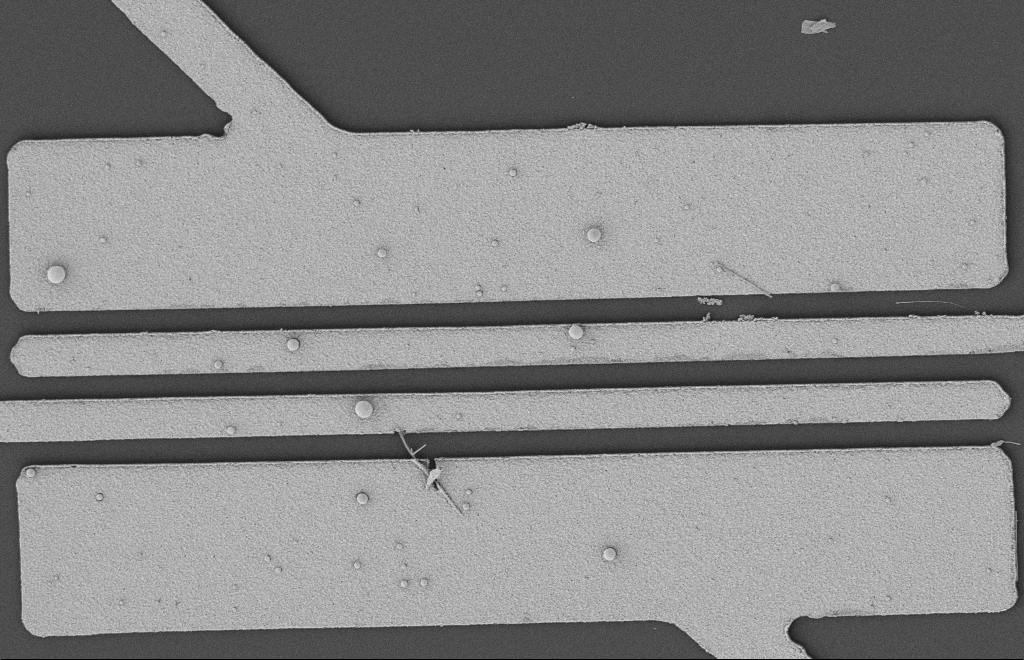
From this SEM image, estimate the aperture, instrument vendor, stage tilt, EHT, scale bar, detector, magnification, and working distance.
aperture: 20 µm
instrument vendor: Zeiss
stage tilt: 0°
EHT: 2 kV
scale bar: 2000 nm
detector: SE2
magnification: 5.96 K X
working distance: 12 mm